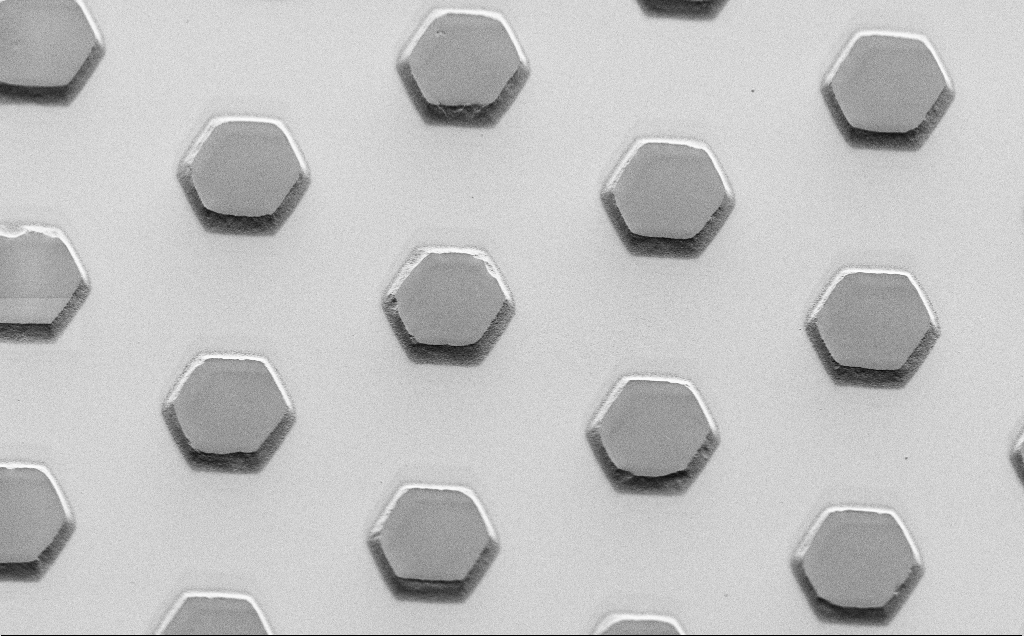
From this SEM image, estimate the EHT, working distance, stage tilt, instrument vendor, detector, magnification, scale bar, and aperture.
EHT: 1.5 kV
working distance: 7 mm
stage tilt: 45°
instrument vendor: Zeiss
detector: SE2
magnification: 2.25 K X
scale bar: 10000 nm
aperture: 30 µm